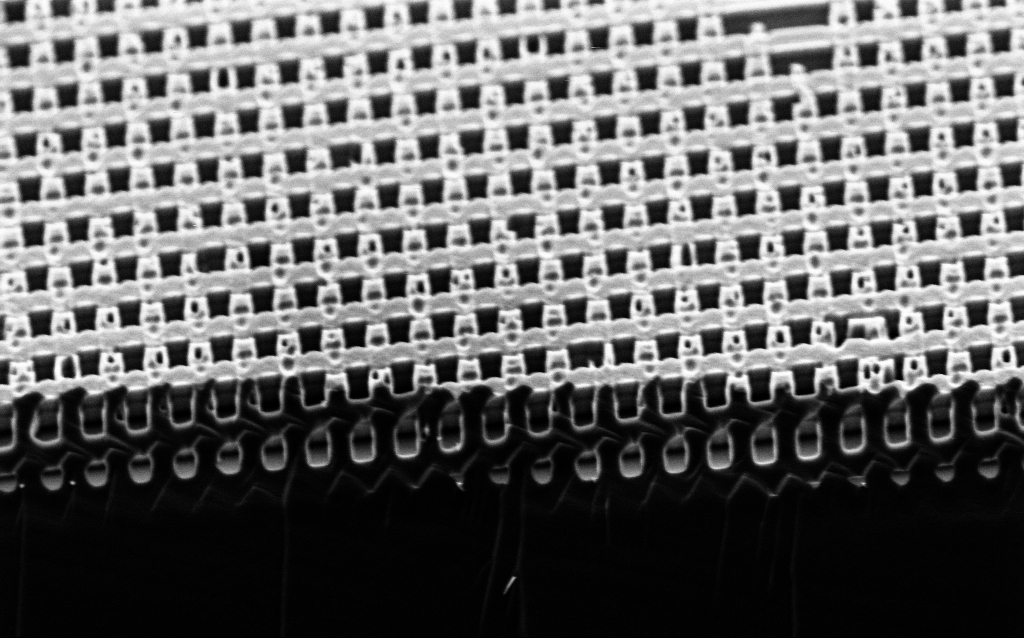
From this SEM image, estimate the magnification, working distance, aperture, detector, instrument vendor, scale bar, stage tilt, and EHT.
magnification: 32.7 K X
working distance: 3 mm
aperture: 30 µm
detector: InLens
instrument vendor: Zeiss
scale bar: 2000 nm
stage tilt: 45°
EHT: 2 kV